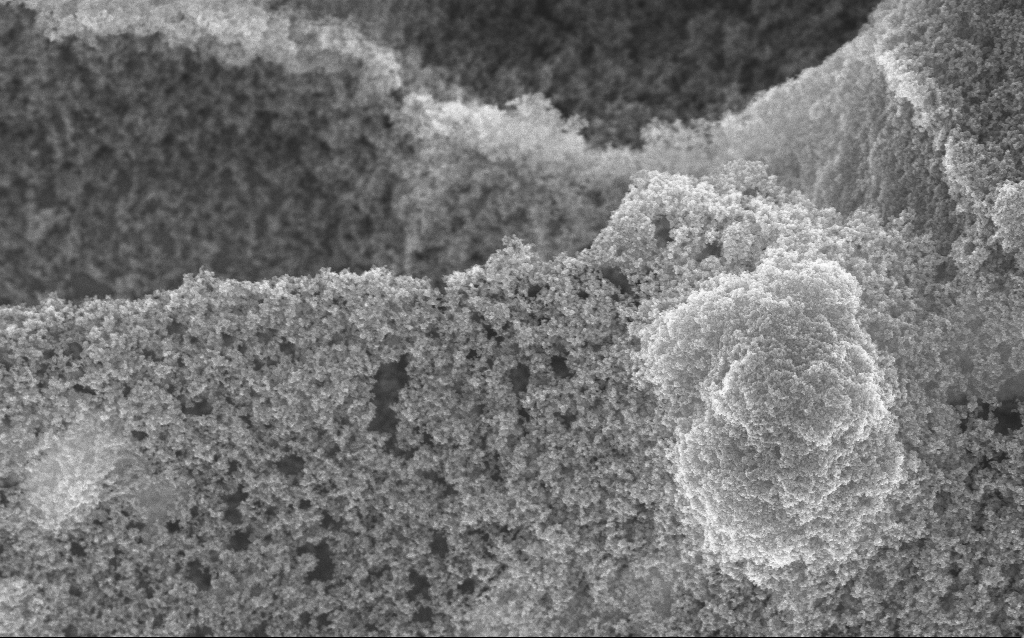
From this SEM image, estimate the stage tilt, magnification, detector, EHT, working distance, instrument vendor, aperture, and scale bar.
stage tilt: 0°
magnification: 37.88 K X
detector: InLens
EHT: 10 kV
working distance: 2.5 mm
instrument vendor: Zeiss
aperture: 30 µm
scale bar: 1000 nm